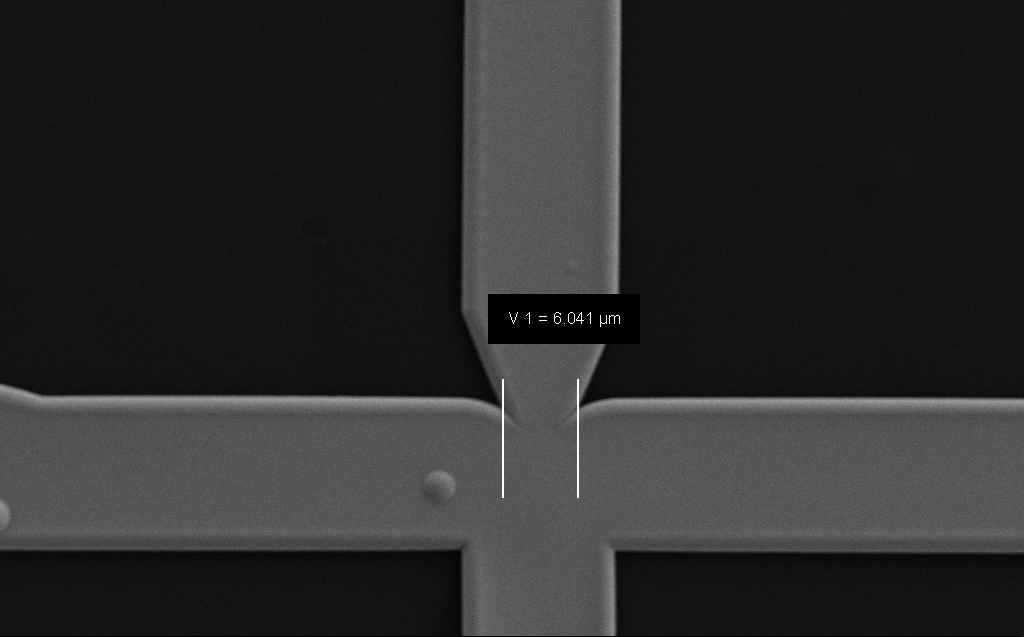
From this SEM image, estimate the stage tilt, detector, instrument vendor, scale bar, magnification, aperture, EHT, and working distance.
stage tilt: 0°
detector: SE2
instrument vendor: Zeiss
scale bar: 10000 nm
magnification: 4.56 K X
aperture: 30 µm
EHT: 1.1 kV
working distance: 6 mm